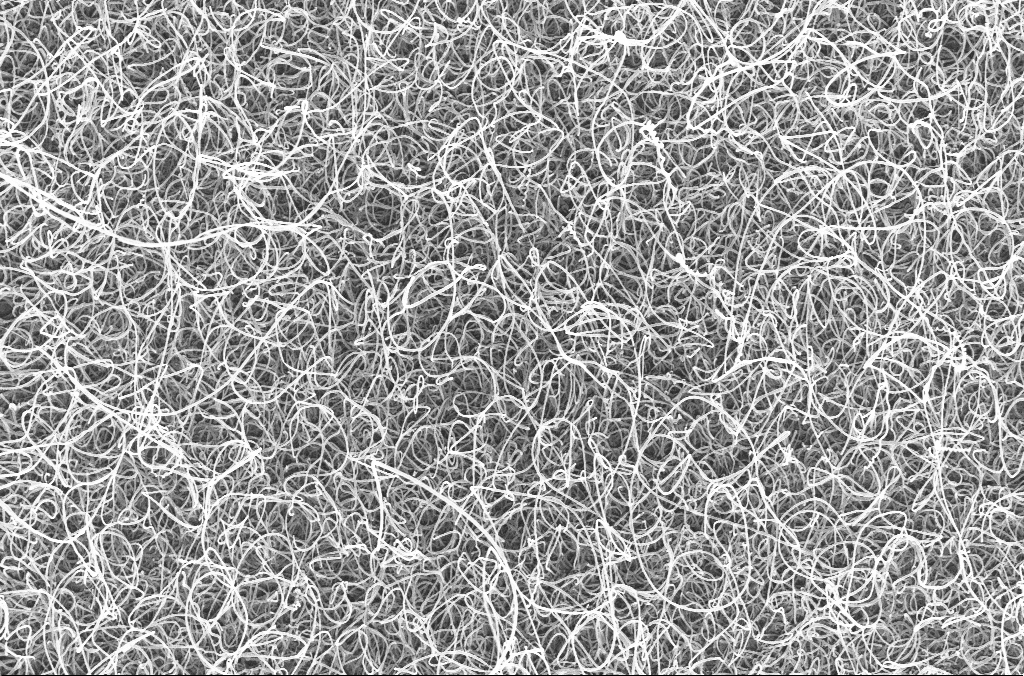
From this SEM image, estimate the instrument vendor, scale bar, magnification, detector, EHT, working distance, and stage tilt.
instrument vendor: Zeiss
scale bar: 1000 nm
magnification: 20 K X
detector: InLens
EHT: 20 kV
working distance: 4.5 mm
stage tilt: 0°